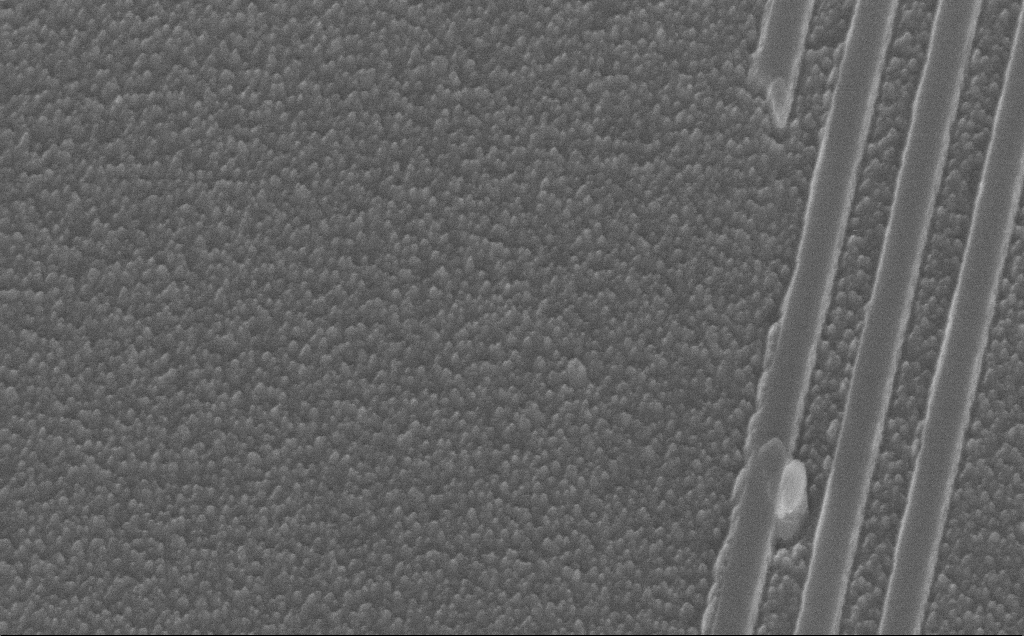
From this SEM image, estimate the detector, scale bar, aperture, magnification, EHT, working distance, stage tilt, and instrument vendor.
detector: InLens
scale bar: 1000 nm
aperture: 30 µm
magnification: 59.54 K X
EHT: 10 kV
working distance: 6 mm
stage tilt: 38°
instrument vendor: Zeiss